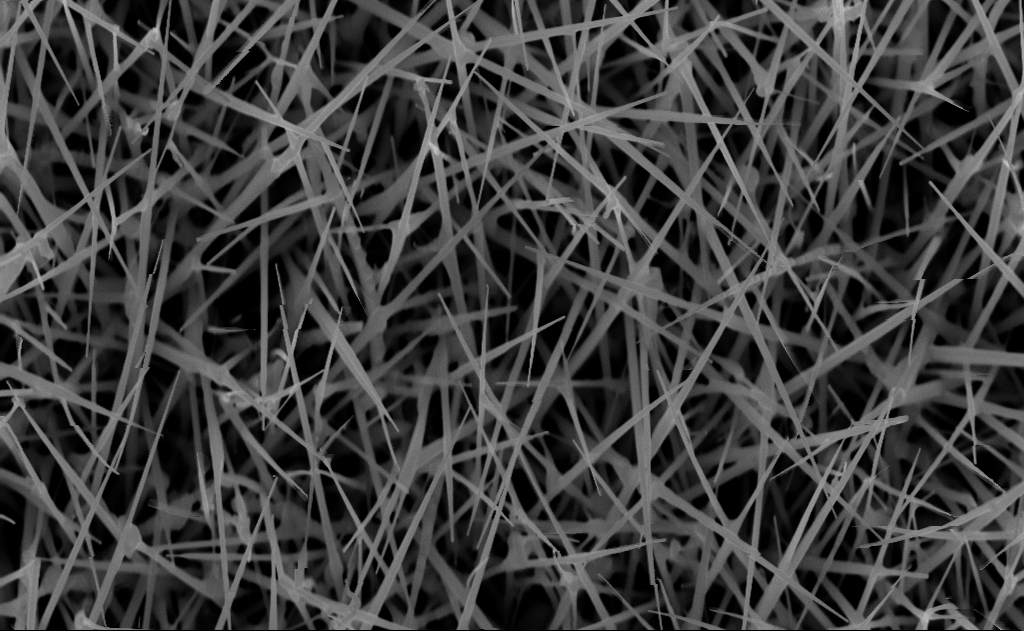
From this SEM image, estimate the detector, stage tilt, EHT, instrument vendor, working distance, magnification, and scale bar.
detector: InLens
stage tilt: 0°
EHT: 10 kV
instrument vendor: Zeiss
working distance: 7 mm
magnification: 40 K X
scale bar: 1000 nm